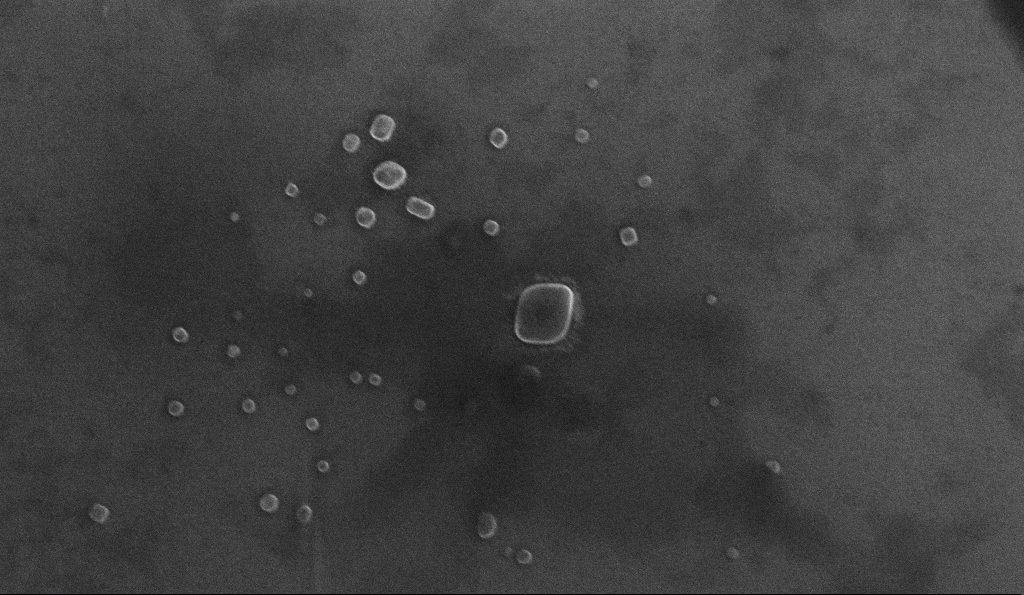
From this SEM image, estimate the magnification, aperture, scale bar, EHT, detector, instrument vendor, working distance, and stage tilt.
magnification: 98.58 K X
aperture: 30 µm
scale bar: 200 nm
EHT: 10 kV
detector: InLens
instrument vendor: Zeiss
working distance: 5.3 mm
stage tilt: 0°